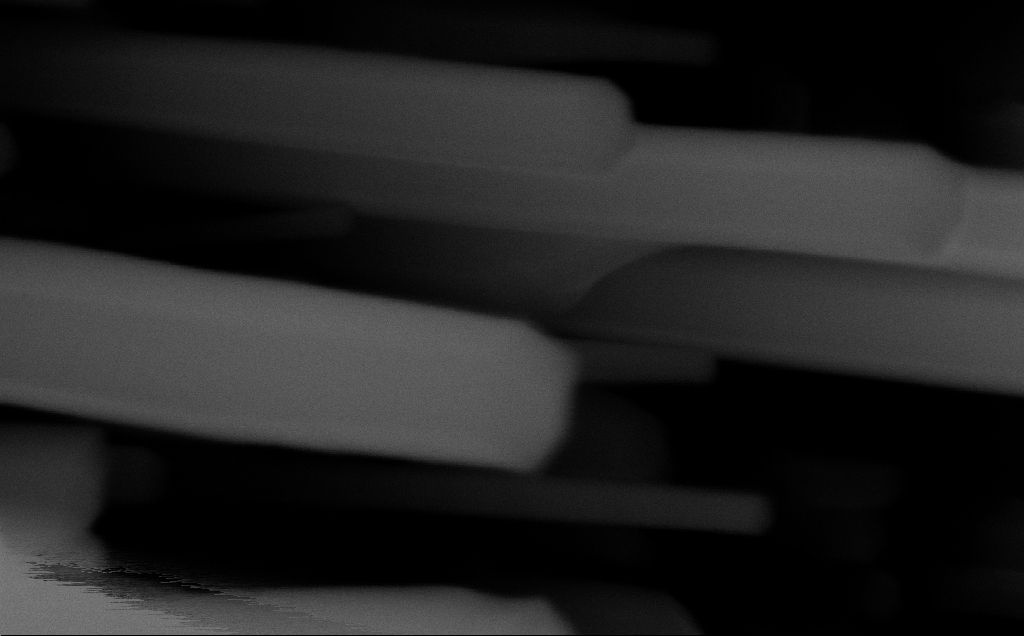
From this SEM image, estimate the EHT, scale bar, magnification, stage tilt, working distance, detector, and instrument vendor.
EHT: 10 kV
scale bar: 200 nm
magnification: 297.84 K X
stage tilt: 0°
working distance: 6 mm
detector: InLens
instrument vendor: Zeiss